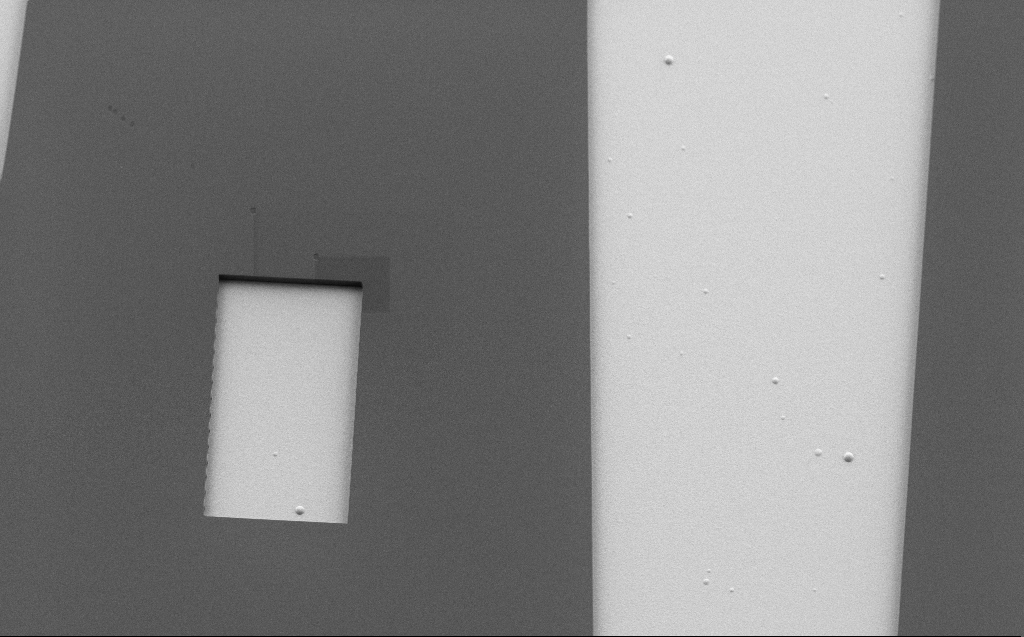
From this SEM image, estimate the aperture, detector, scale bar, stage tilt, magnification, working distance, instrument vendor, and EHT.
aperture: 30 µm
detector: SE2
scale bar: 10000 nm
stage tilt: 30°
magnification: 1.79 K X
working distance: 6 mm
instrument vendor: Zeiss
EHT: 1.1 kV